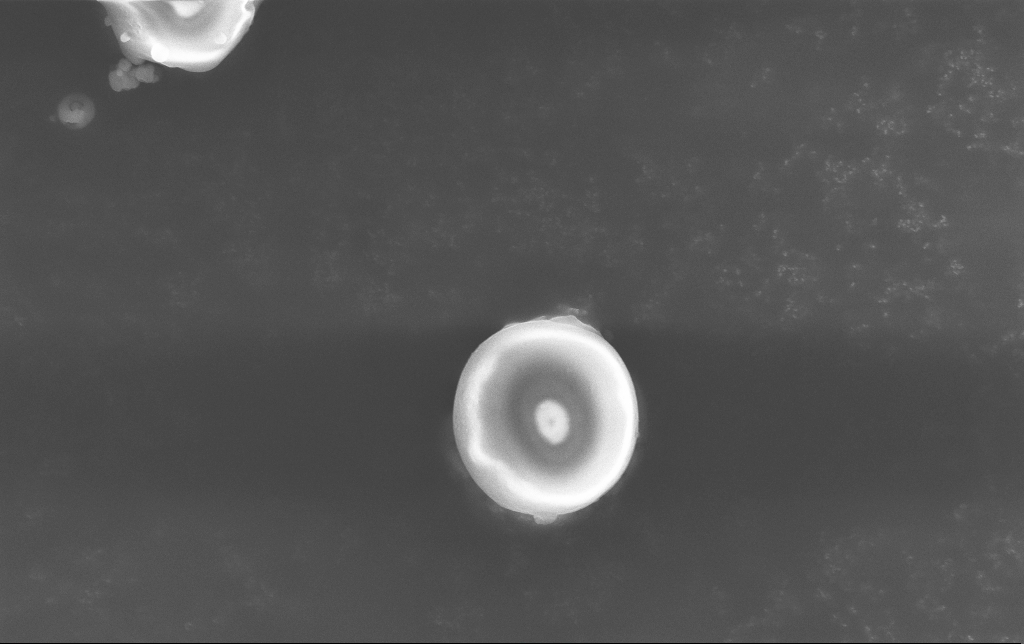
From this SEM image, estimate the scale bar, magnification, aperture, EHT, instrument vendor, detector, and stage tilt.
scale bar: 2000 nm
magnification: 7.97 K X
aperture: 30 µm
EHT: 15 kV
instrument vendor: Zeiss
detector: InLens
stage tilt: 0°